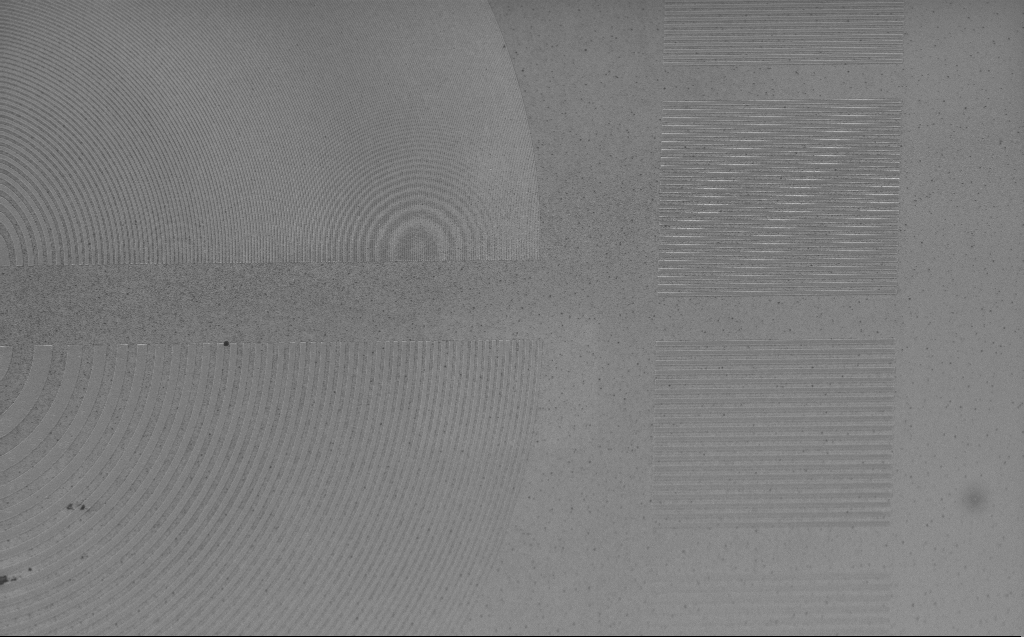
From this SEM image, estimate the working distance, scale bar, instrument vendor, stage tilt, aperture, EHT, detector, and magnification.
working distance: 4 mm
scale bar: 10000 nm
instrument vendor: Zeiss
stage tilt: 30°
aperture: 30 µm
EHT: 5 kV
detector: InLens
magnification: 2.98 K X